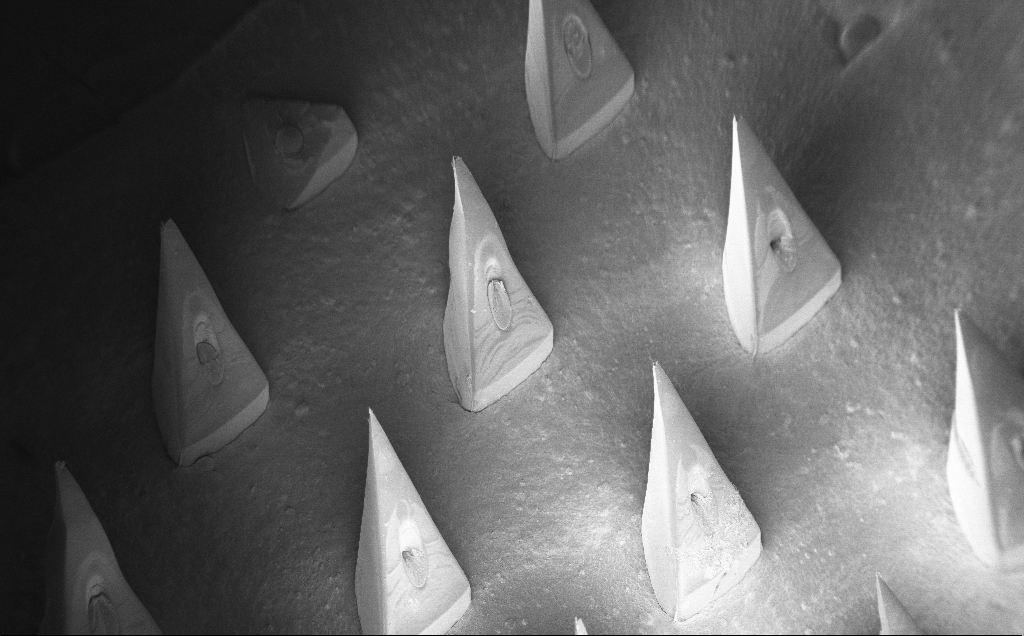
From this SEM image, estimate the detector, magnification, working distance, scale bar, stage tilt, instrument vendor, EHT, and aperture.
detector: InLens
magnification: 0.073 K X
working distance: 10 mm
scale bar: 200000 nm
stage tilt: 40°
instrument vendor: Zeiss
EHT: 5 kV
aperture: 30 µm